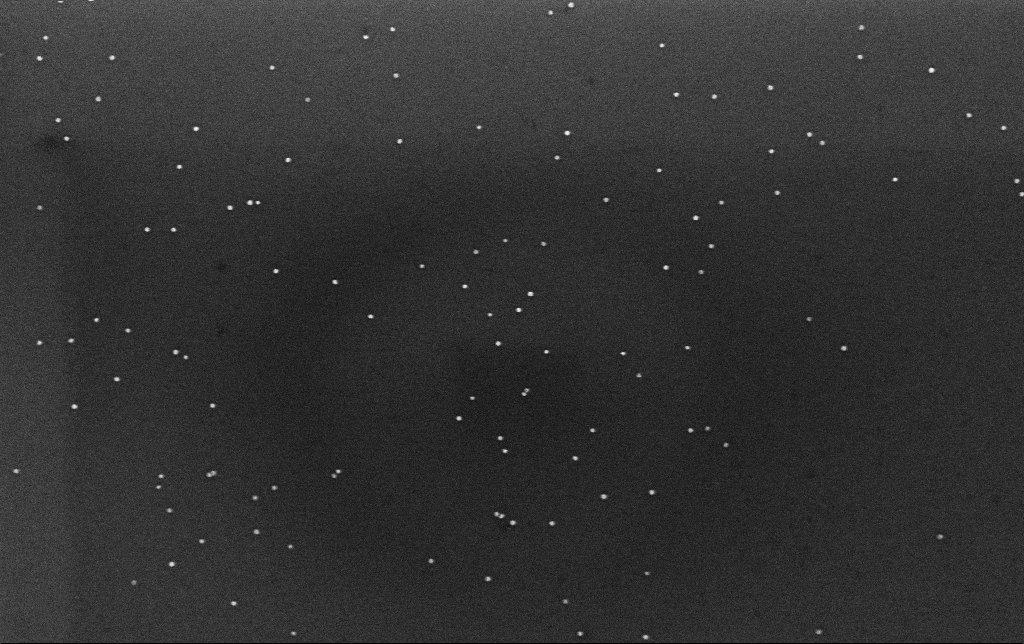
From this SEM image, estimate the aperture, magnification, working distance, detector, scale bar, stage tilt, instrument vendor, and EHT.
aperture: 30 µm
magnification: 100 K X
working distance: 3.1 mm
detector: InLens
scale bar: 200 nm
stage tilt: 0°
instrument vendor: Zeiss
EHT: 10 kV